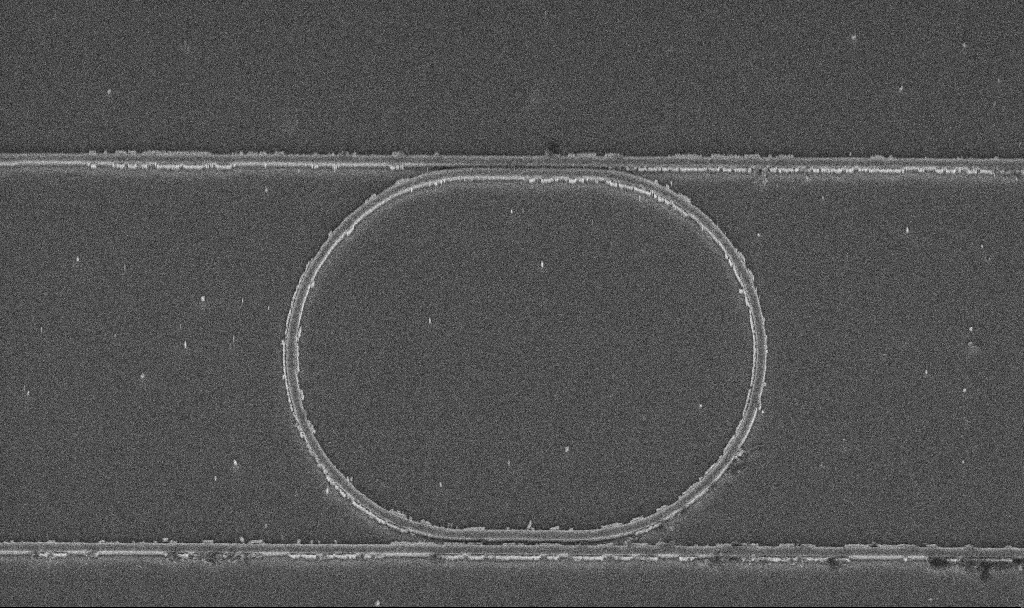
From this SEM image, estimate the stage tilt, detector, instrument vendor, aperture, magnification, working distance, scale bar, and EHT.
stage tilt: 45°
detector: InLens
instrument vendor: Zeiss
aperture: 30 µm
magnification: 6.79 K X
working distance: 9.5 mm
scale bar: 10000 nm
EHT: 5 kV